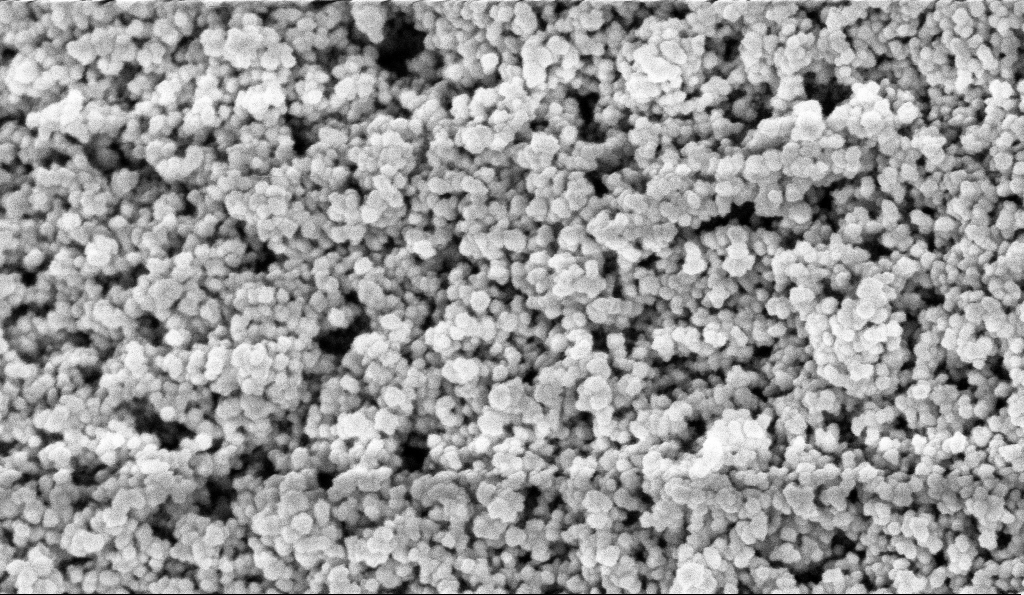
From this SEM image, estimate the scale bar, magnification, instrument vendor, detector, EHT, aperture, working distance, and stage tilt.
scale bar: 200 nm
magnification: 135 K X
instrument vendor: Zeiss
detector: InLens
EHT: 5 kV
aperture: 30 µm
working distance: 5.9 mm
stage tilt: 0°